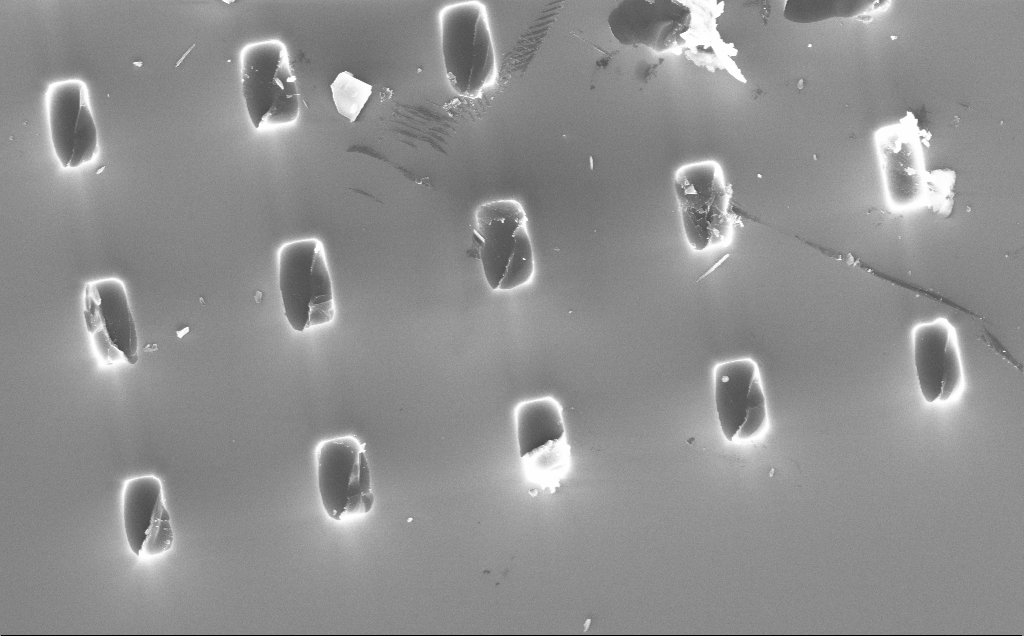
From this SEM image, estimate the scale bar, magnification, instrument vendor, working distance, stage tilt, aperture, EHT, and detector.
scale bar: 2000 nm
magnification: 7.53 K X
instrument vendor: Zeiss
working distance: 3 mm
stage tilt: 0.1°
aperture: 30 µm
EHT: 10 kV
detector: InLens